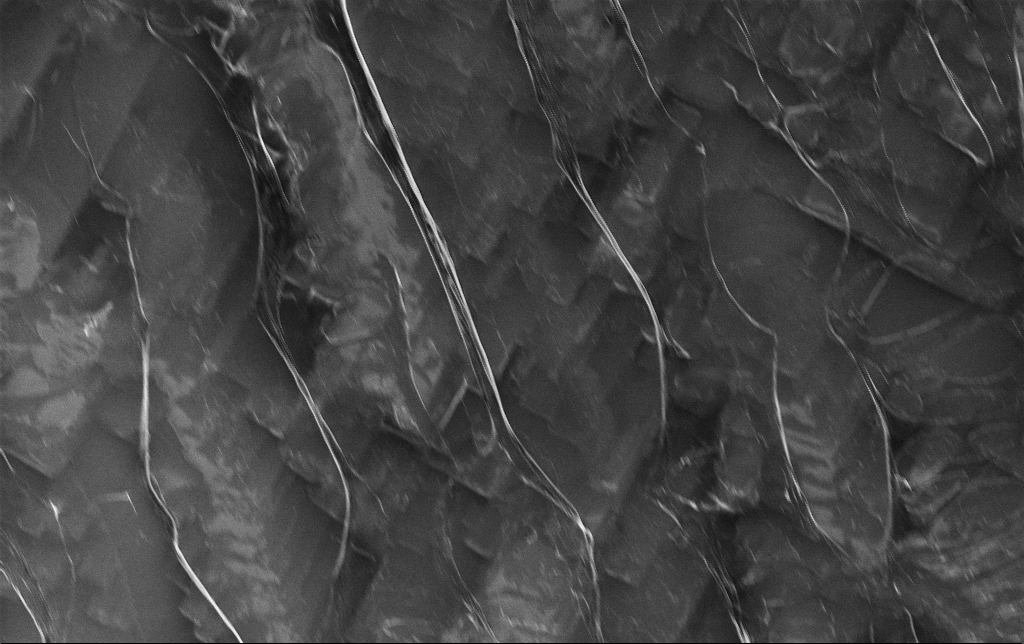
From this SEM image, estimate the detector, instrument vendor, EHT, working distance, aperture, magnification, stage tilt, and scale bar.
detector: InLens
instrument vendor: Zeiss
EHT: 5 kV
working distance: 3 mm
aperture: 30 µm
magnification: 1.96 K X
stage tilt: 0°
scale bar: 20000 nm